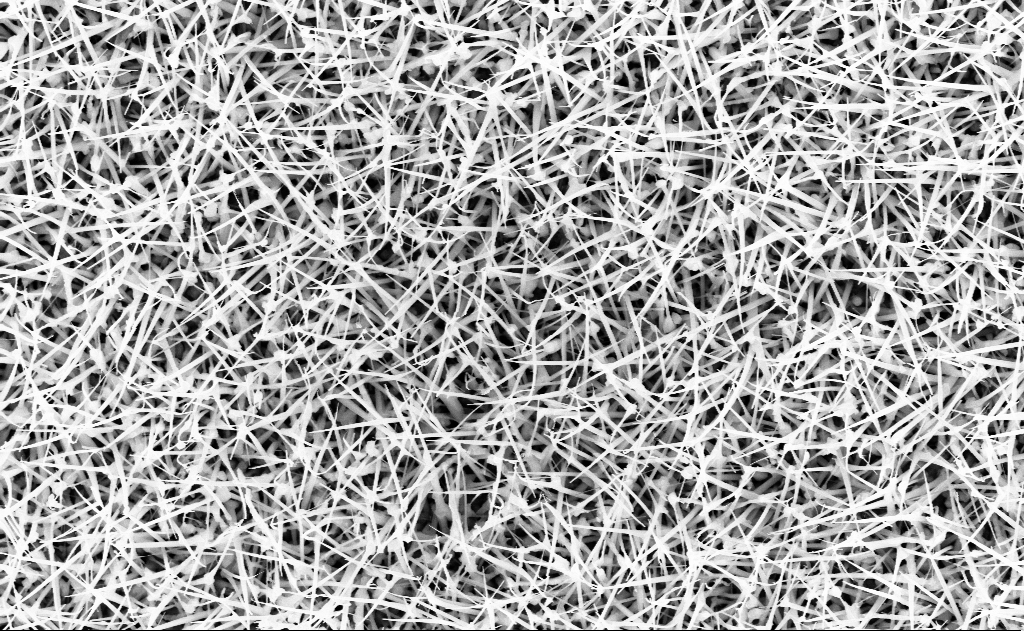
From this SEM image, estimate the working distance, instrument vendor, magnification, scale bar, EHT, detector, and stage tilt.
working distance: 13 mm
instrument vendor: Zeiss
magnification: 20 K X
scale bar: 1000 nm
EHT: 10 kV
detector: InLens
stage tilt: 0°